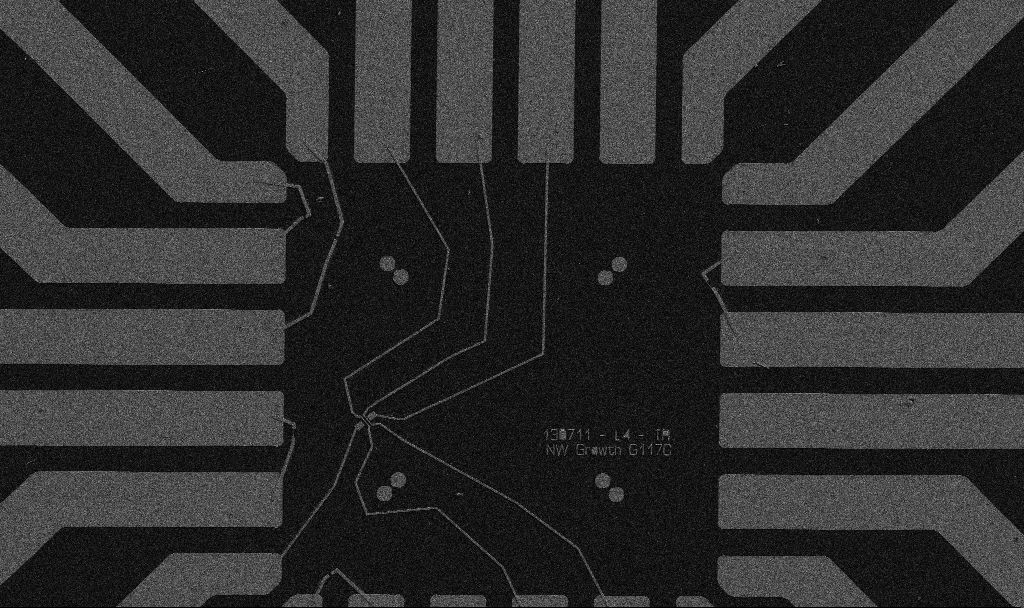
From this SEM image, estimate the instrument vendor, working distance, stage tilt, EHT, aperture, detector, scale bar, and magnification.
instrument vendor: Zeiss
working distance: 10.7 mm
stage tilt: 0°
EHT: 5 kV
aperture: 30 µm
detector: SE2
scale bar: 20000 nm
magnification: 1 K X